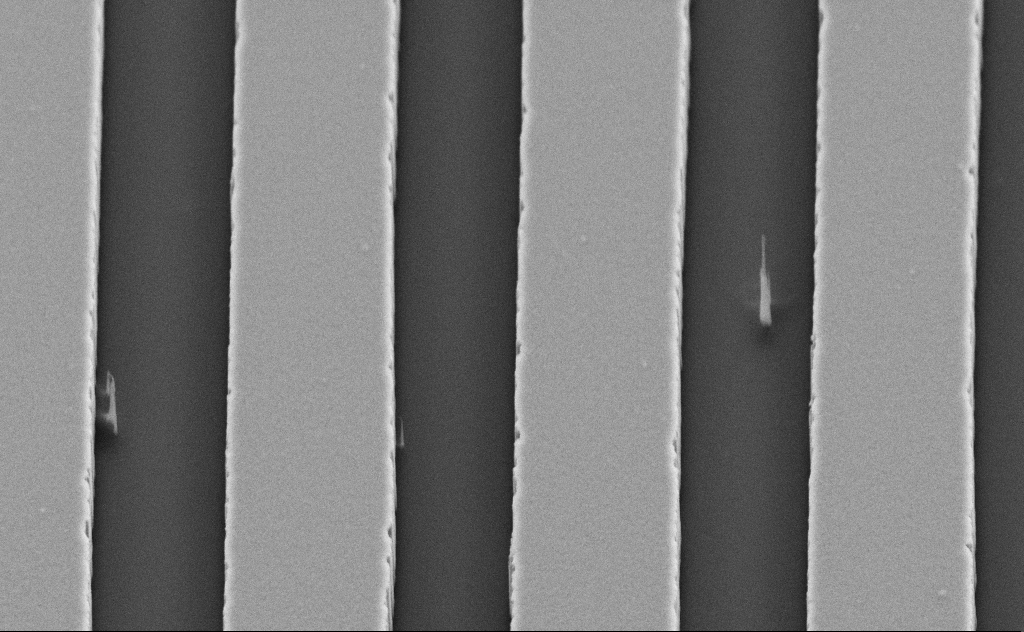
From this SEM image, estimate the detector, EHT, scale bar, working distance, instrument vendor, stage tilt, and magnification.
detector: SE2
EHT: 5 kV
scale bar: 2000 nm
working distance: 9 mm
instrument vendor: Zeiss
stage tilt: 45°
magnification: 26.72 K X